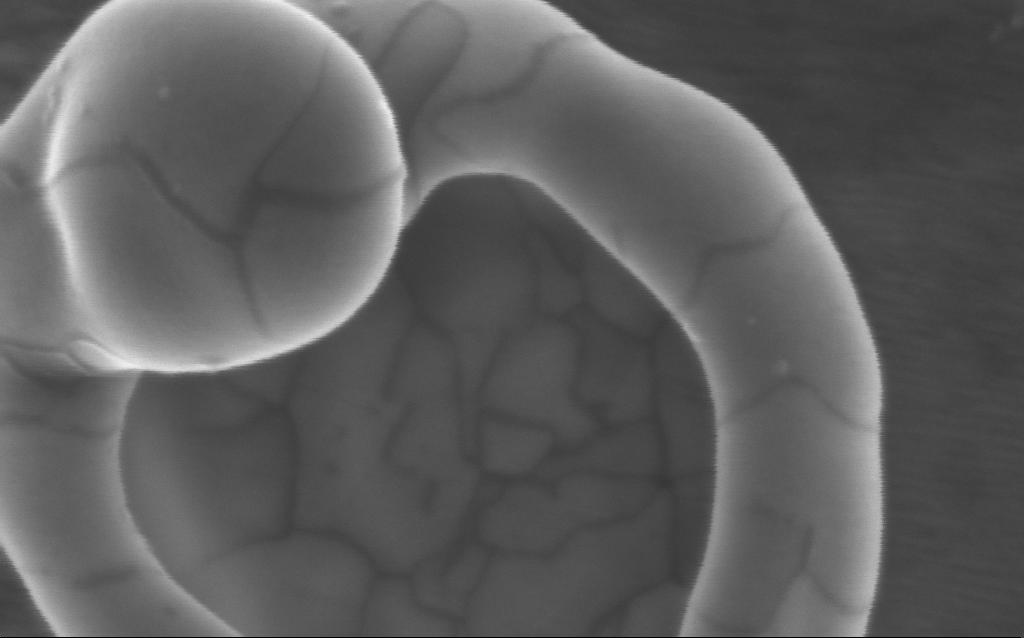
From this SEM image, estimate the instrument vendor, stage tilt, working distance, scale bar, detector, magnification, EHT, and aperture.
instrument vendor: Zeiss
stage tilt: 0°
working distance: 4 mm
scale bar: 200 nm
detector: InLens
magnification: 359.02 K X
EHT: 5 kV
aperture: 30 µm